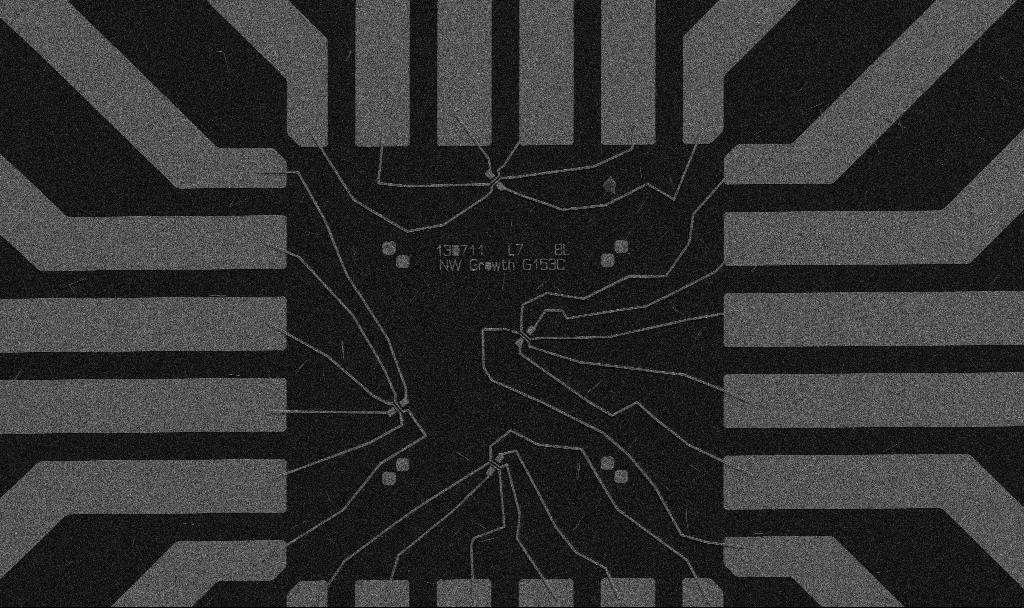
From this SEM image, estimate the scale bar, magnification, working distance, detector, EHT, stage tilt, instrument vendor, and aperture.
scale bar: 20000 nm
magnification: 1 K X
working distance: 10.7 mm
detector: SE2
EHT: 5 kV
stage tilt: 0°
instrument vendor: Zeiss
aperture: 30 µm